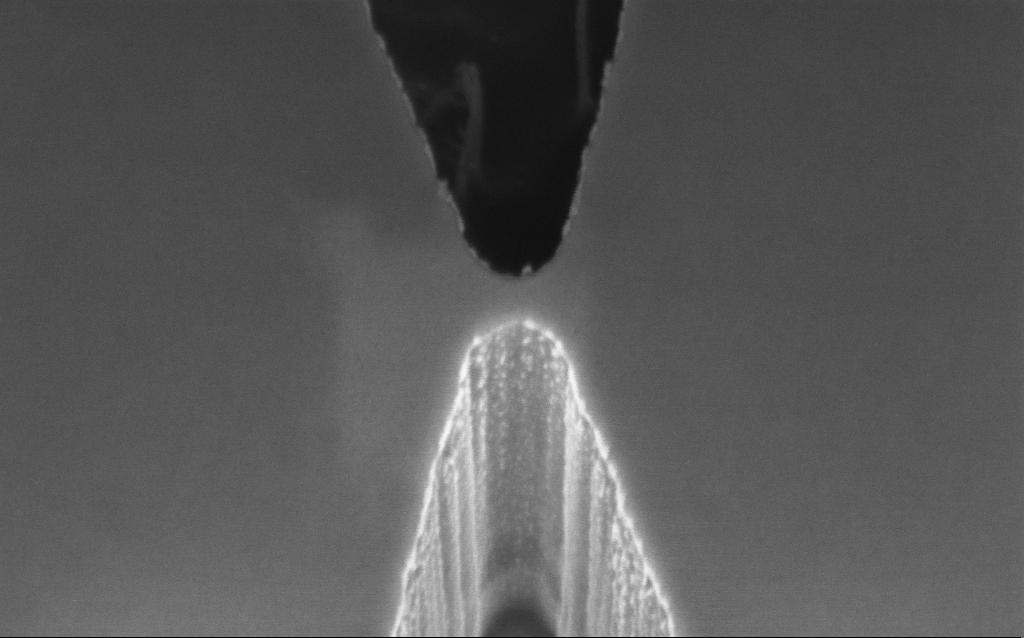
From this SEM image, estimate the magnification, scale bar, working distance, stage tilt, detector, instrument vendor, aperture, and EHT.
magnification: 169.59 K X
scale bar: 200 nm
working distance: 6 mm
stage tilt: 45°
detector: InLens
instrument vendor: Zeiss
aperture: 30 µm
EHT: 2 kV